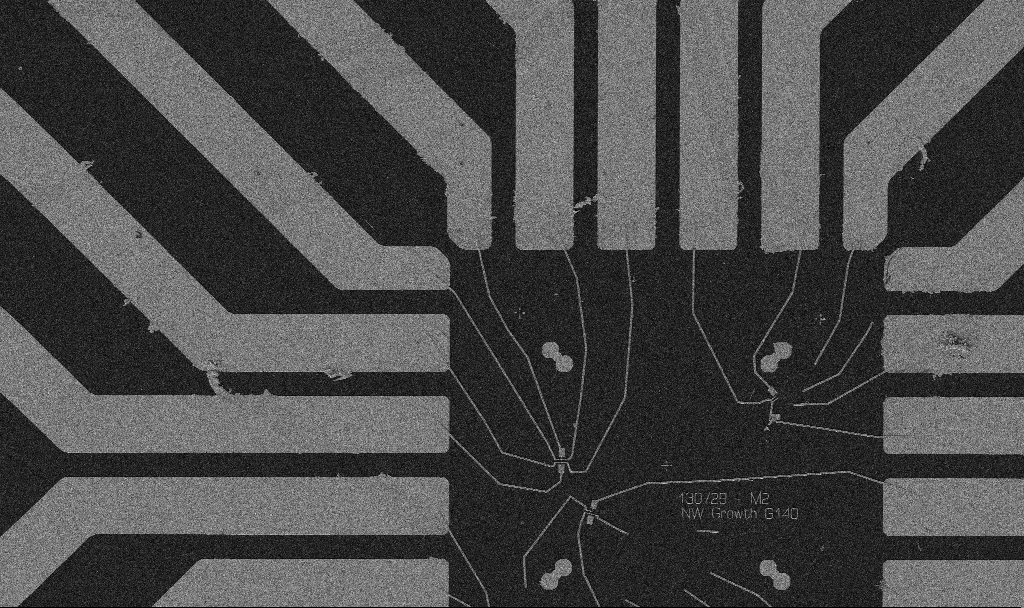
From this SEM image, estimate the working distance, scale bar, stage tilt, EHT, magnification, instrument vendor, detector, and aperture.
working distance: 10.7 mm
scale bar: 20000 nm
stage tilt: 0°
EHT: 5 kV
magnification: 1 K X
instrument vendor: Zeiss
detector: SE2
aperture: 30 µm